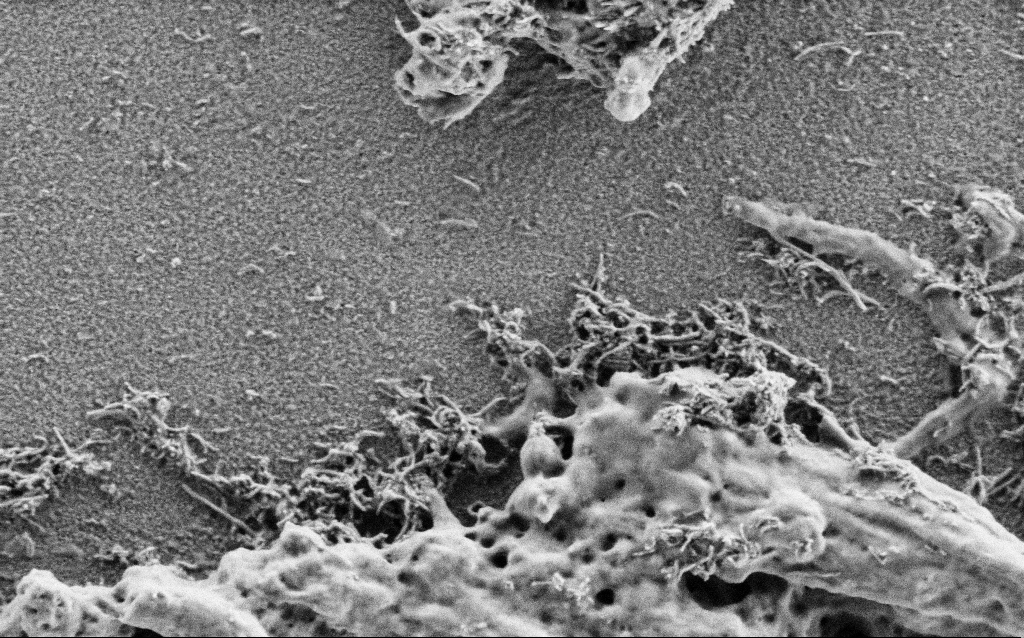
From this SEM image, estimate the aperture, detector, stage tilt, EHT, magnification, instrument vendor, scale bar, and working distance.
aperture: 30 µm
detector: SE2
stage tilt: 0°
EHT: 1 kV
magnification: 50 K X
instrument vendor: Zeiss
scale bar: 1000 nm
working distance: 4 mm